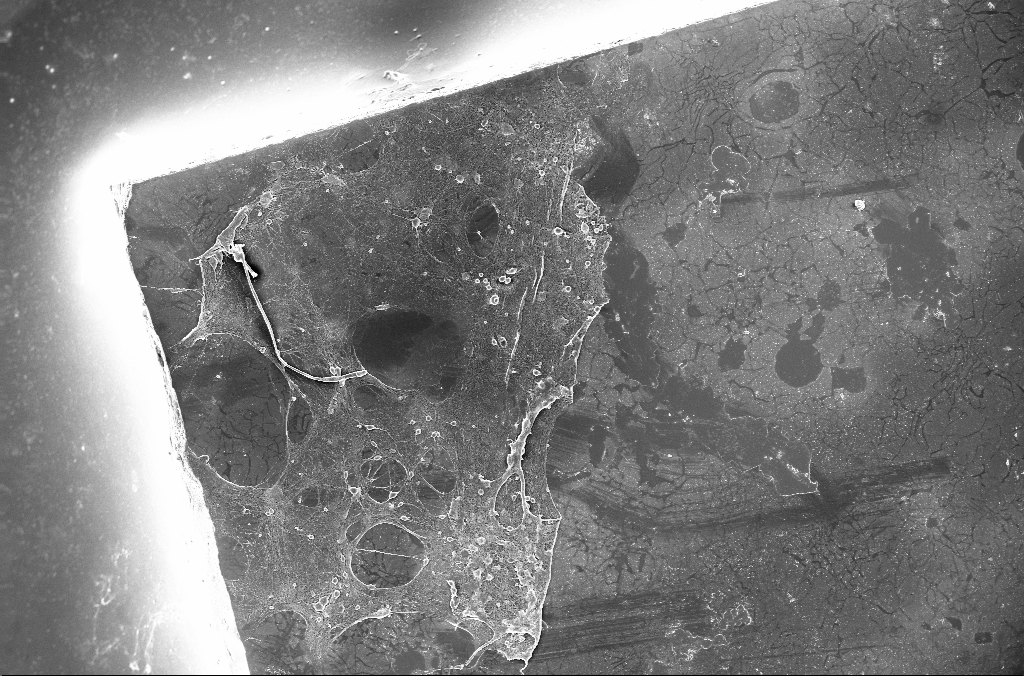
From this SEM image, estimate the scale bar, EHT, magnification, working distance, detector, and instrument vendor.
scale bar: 100000 nm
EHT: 15 kV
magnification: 0.2 K X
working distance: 3.1 mm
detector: InLens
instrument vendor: Zeiss